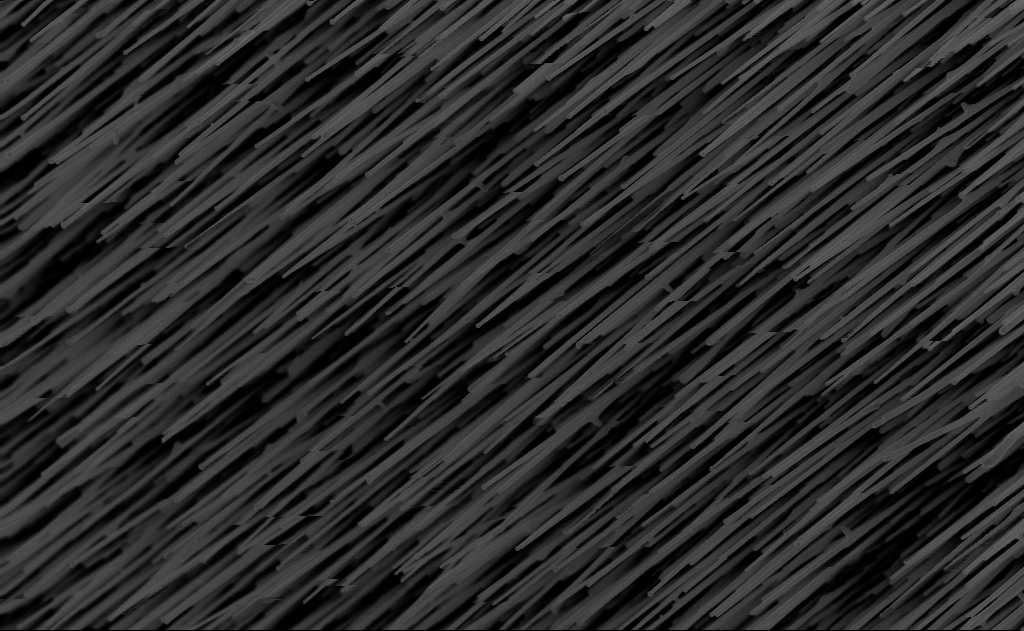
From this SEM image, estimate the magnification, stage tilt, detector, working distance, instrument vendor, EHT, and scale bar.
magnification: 20 K X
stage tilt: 0°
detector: InLens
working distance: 10 mm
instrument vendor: Zeiss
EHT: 10 kV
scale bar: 2000 nm